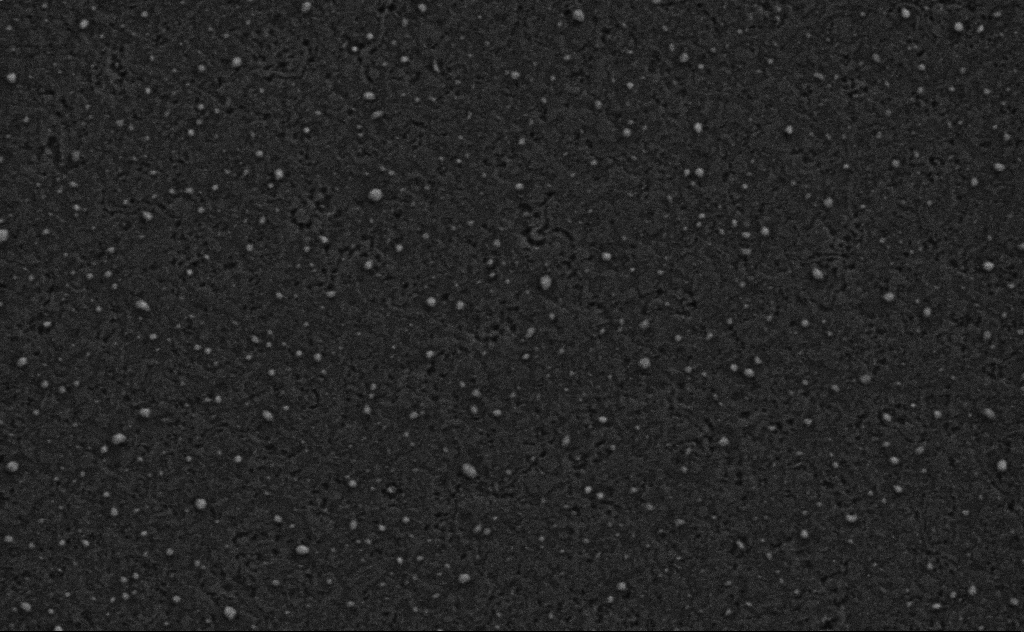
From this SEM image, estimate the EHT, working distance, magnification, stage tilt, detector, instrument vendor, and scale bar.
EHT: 3 kV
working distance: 4 mm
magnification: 80 K X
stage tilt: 0°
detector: SE2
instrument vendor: Zeiss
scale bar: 200 nm